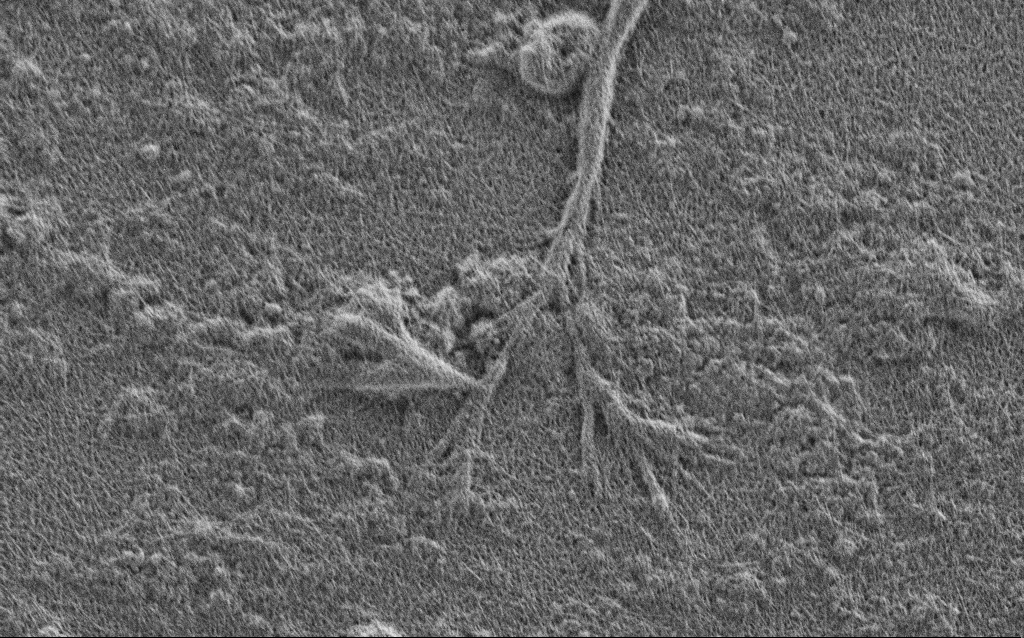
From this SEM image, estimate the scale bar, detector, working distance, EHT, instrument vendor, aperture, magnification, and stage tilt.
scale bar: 2000 nm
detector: SE2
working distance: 4 mm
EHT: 1 kV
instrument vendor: Zeiss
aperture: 30 µm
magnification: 10 K X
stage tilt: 0°